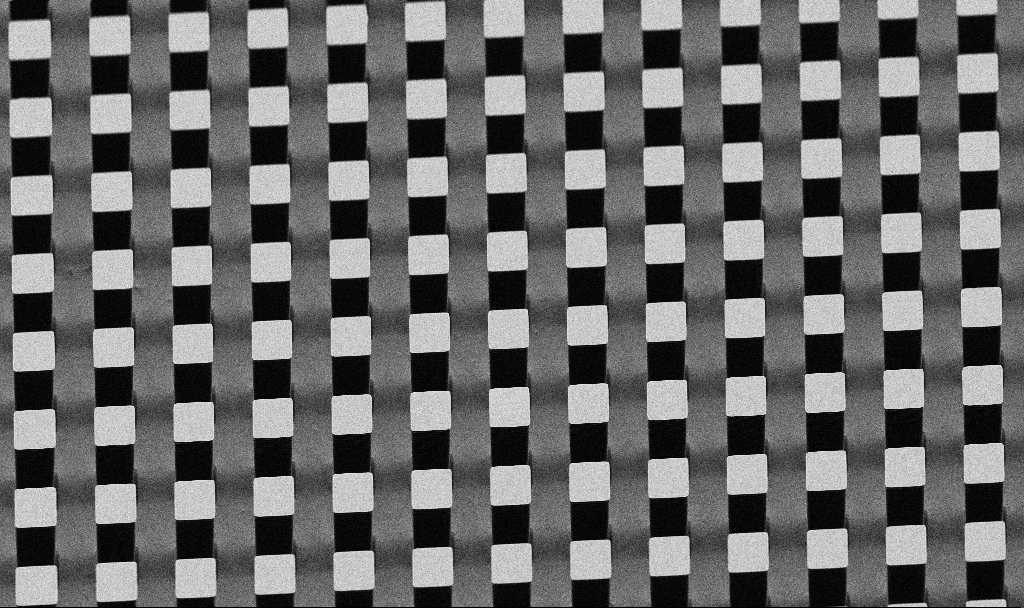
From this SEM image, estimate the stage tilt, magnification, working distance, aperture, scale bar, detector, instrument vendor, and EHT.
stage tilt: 45°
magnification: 1.82 K X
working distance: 7.5 mm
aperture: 30 µm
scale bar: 20000 nm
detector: SE2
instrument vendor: Zeiss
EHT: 5 kV